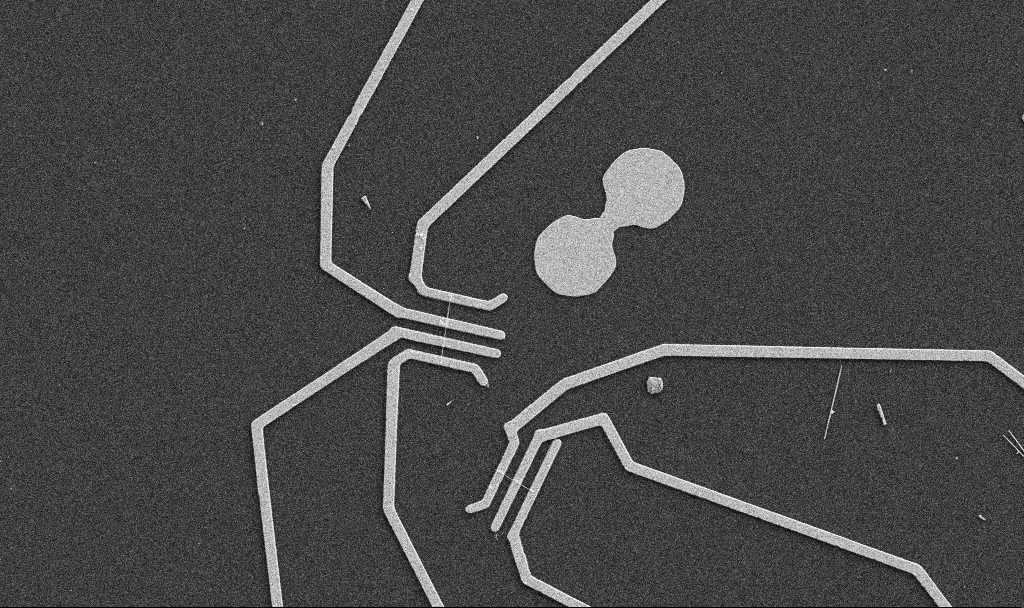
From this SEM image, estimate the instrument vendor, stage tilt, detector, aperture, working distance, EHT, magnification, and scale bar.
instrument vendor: Zeiss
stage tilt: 0°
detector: SE2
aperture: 30 µm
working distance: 10.7 mm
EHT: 5 kV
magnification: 5 K X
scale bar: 10000 nm